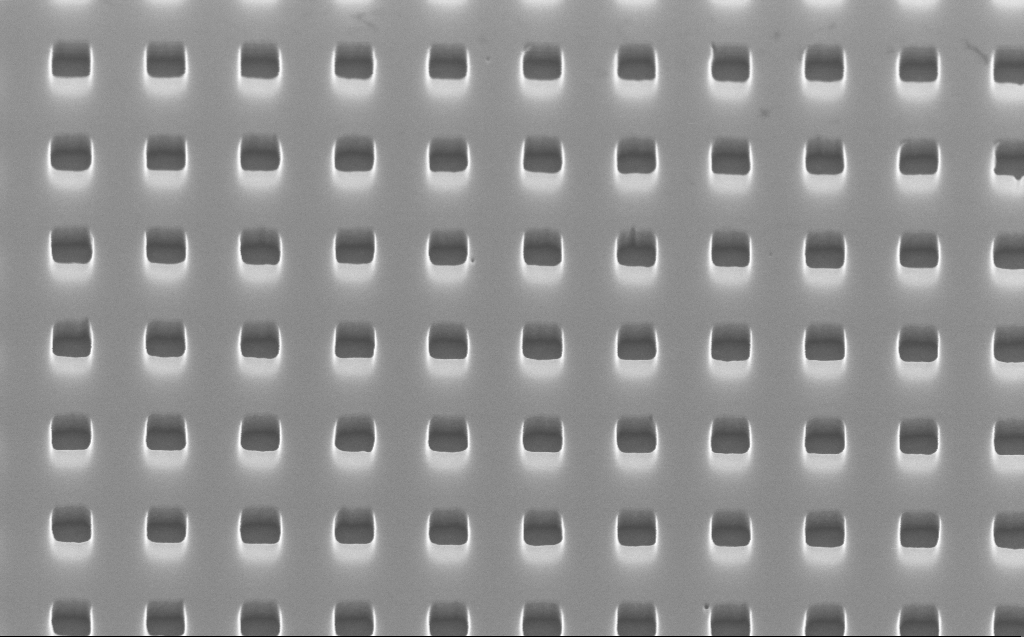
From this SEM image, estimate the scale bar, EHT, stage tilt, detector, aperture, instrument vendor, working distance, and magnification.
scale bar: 1000 nm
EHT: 10 kV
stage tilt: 45°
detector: InLens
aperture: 30 µm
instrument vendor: Zeiss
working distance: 3 mm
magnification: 70 K X